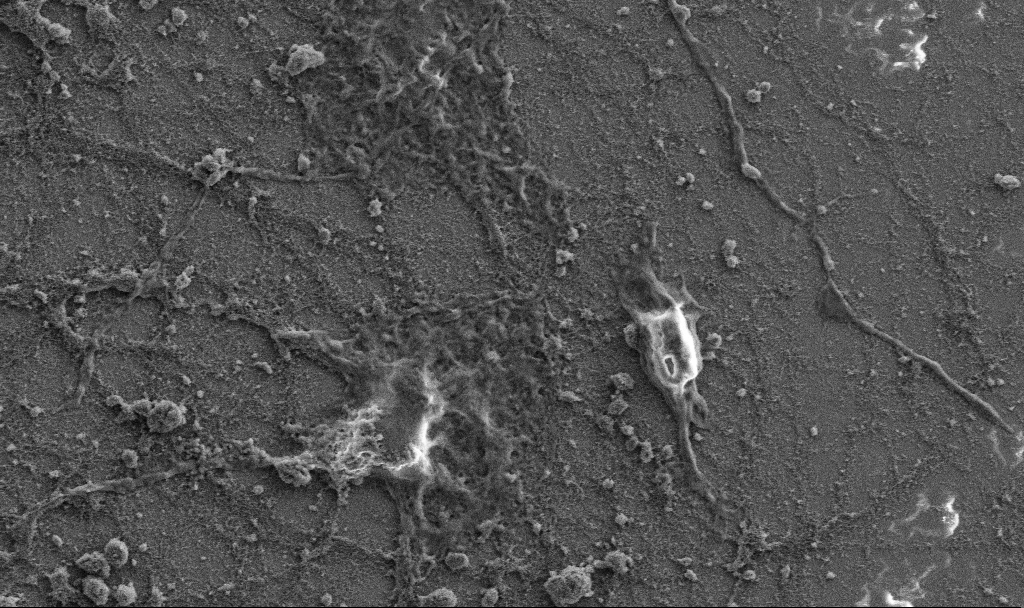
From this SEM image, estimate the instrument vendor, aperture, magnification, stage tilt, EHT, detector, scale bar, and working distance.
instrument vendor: Zeiss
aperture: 30 µm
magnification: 3.5 K X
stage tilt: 0°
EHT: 5 kV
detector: SE2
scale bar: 20000 nm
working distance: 6.8 mm